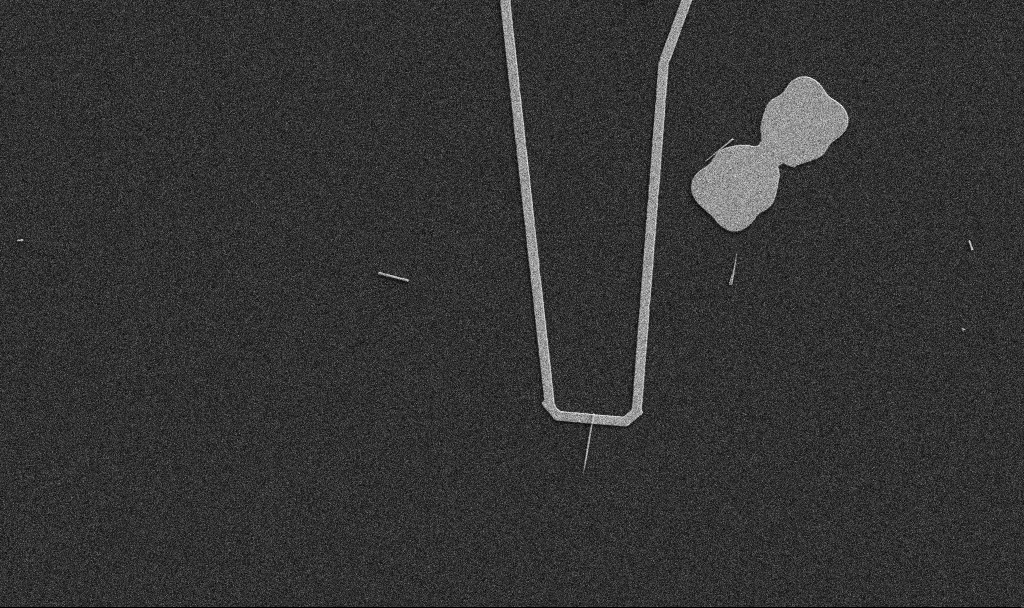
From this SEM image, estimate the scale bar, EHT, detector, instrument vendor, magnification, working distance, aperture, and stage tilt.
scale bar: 10000 nm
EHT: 5 kV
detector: SE2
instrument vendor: Zeiss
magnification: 5 K X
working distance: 10.7 mm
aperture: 30 µm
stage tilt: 0°